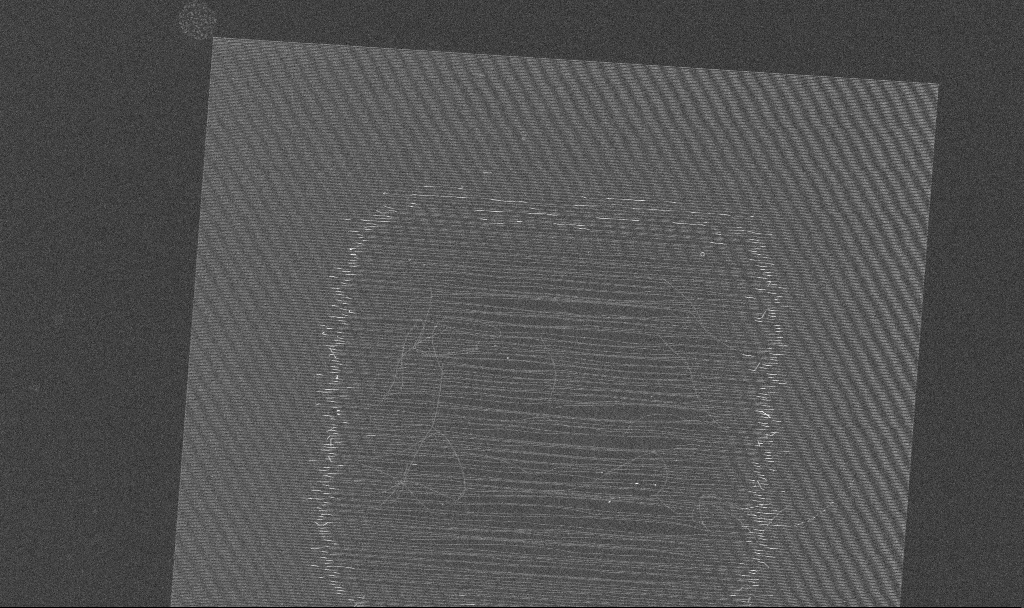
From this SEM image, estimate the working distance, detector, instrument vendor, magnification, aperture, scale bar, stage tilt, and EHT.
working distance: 4.7 mm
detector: InLens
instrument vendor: Zeiss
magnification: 1.79 K X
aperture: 30 µm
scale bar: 20000 nm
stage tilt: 0°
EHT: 5 kV